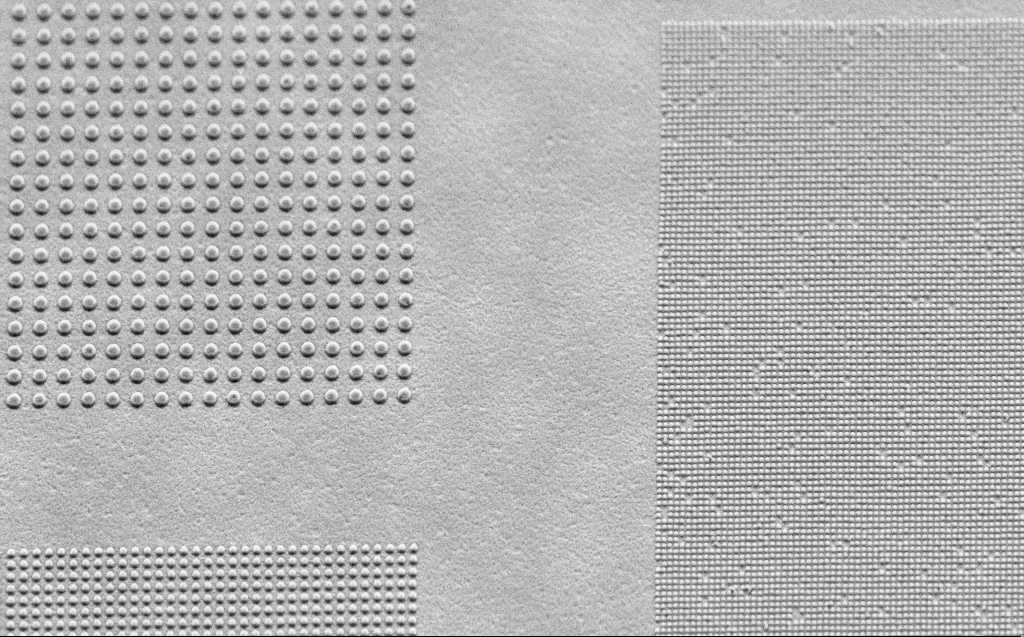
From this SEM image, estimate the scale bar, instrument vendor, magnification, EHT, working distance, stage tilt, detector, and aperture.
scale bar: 2000 nm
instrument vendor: Zeiss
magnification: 11.25 K X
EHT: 2.5 kV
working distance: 6 mm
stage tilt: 44.1°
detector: SE2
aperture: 30 µm